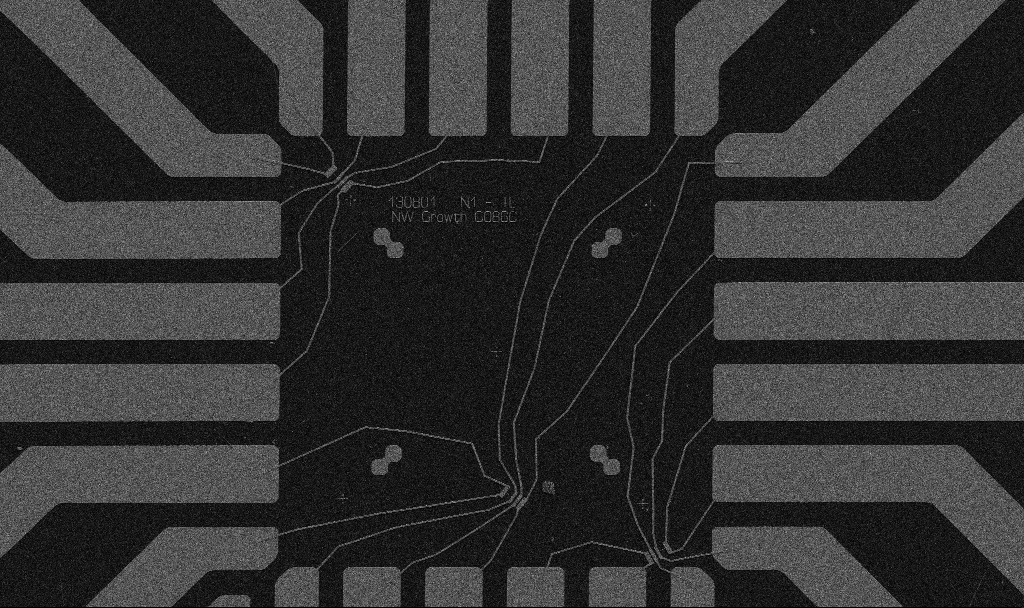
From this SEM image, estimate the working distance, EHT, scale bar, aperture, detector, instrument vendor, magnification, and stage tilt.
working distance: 10.7 mm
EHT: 5 kV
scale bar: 20000 nm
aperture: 30 µm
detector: SE2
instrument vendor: Zeiss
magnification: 1 K X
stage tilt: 0°